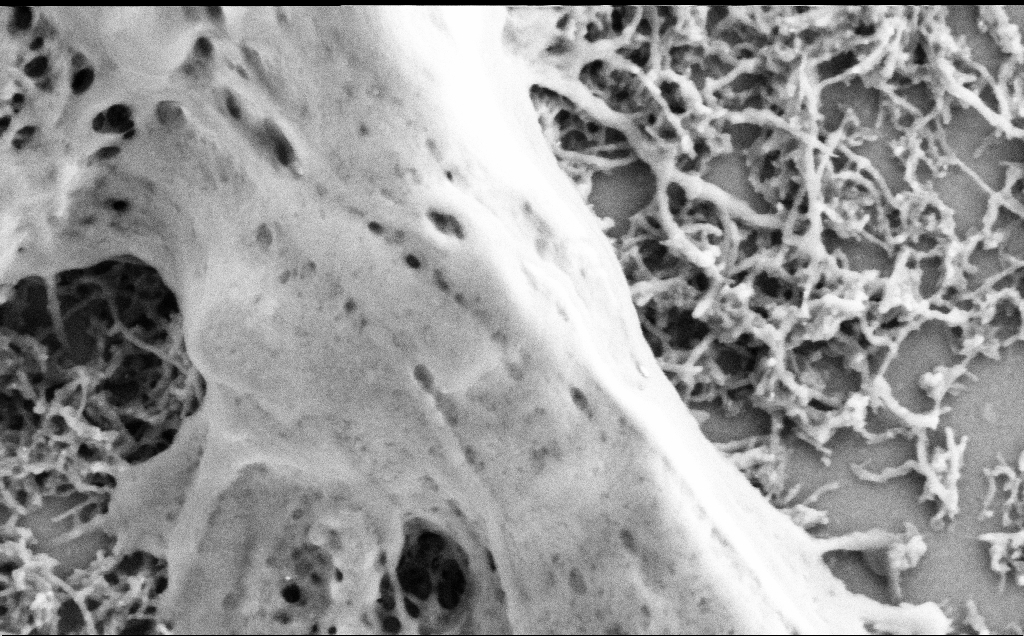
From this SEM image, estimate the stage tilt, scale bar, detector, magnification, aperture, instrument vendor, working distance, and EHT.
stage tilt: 0°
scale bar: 200 nm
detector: SE2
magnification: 75 K X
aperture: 30 µm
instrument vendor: Zeiss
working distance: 7.1 mm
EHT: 2 kV